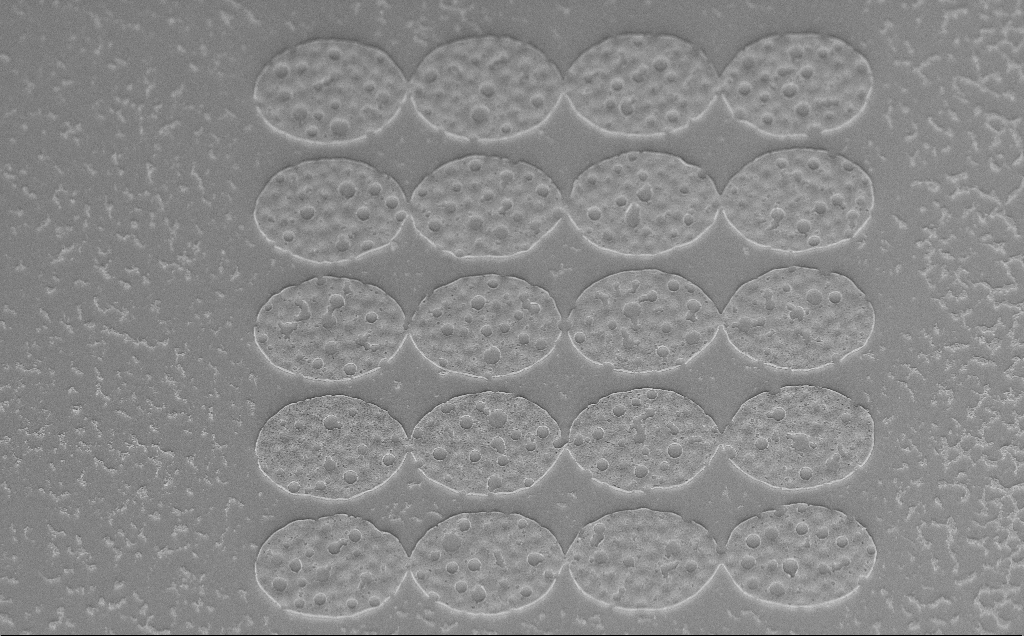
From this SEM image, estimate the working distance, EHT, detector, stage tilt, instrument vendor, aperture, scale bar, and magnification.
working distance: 6 mm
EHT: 5 kV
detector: InLens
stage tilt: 45°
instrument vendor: Zeiss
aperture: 30 µm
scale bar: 10000 nm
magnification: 5.77 K X